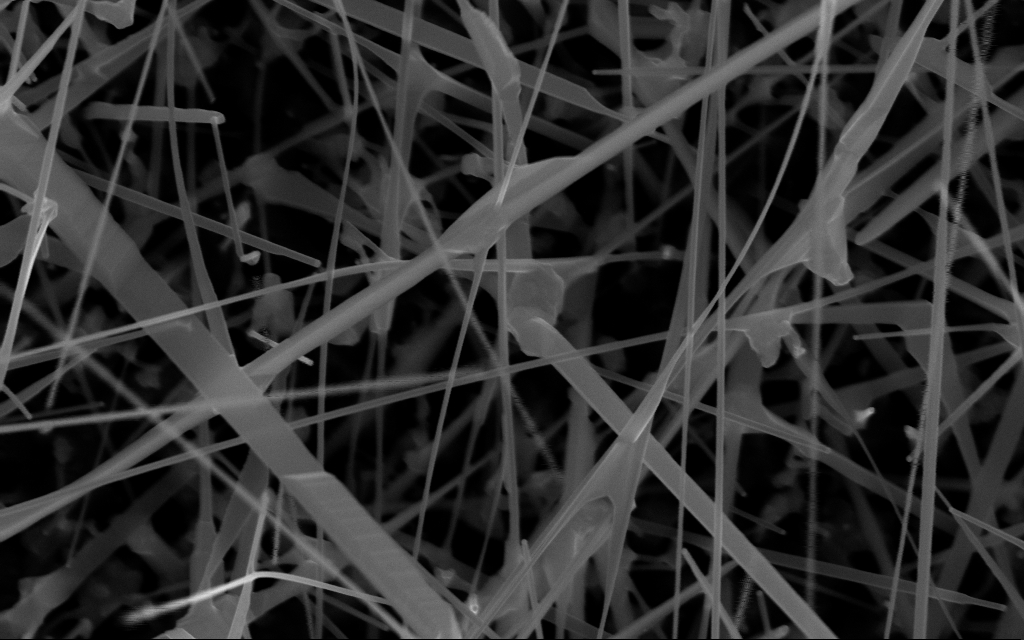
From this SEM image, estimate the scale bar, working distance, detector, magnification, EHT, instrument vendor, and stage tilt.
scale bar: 200 nm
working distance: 7 mm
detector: InLens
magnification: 80 K X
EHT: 10 kV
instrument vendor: Zeiss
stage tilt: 0°